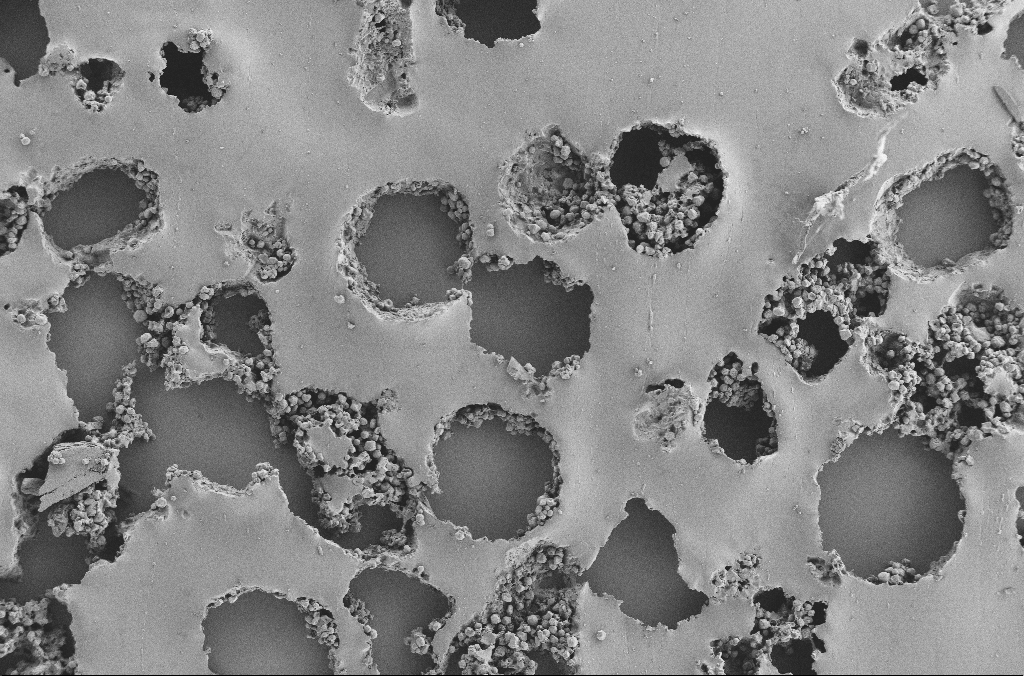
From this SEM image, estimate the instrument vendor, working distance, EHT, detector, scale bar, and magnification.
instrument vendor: Zeiss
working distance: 3.7 mm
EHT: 2 kV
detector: SE2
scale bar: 100000 nm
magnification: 0.25 K X